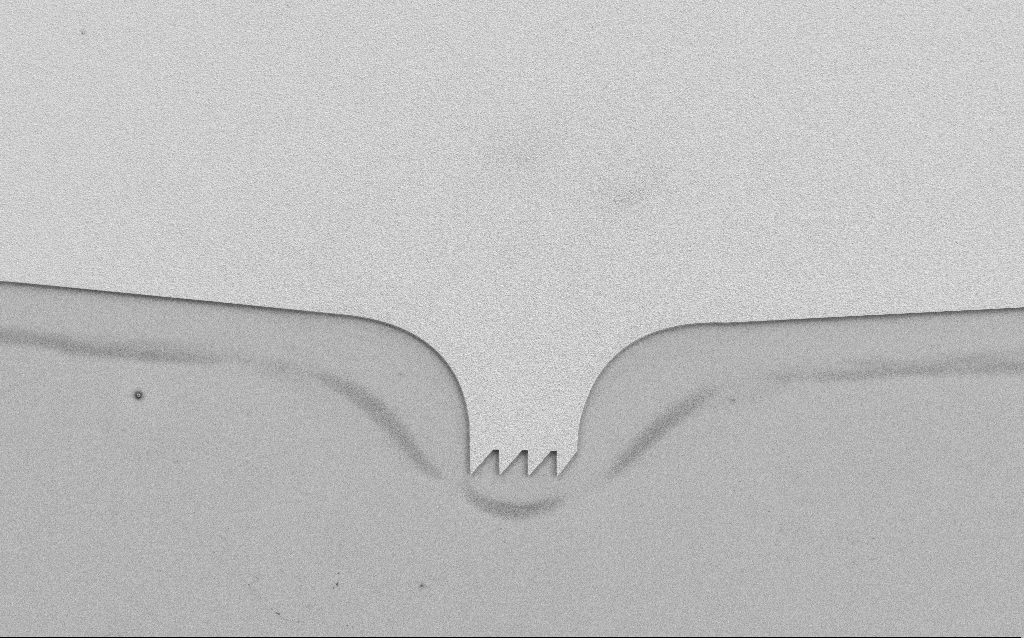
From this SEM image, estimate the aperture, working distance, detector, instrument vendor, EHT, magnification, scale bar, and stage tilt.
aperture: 30 µm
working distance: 4.5 mm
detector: SE2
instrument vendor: Zeiss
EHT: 3 kV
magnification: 0.937 K X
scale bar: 20000 nm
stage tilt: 0°